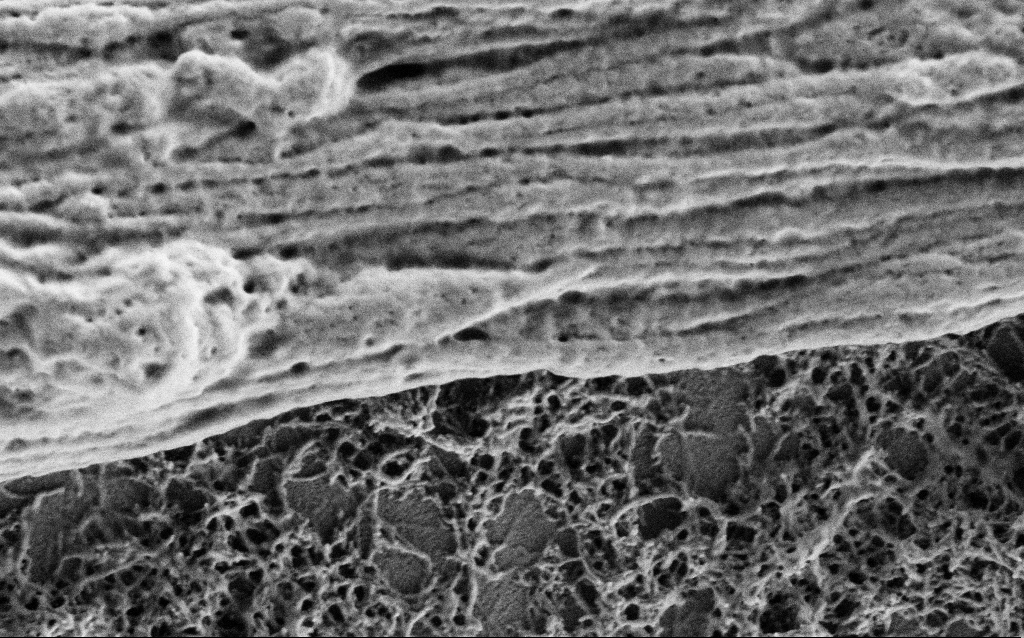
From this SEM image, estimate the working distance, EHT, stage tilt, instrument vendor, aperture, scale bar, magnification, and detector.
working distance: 4 mm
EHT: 1 kV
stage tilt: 0°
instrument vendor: Zeiss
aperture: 30 µm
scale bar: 1000 nm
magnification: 50 K X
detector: SE2